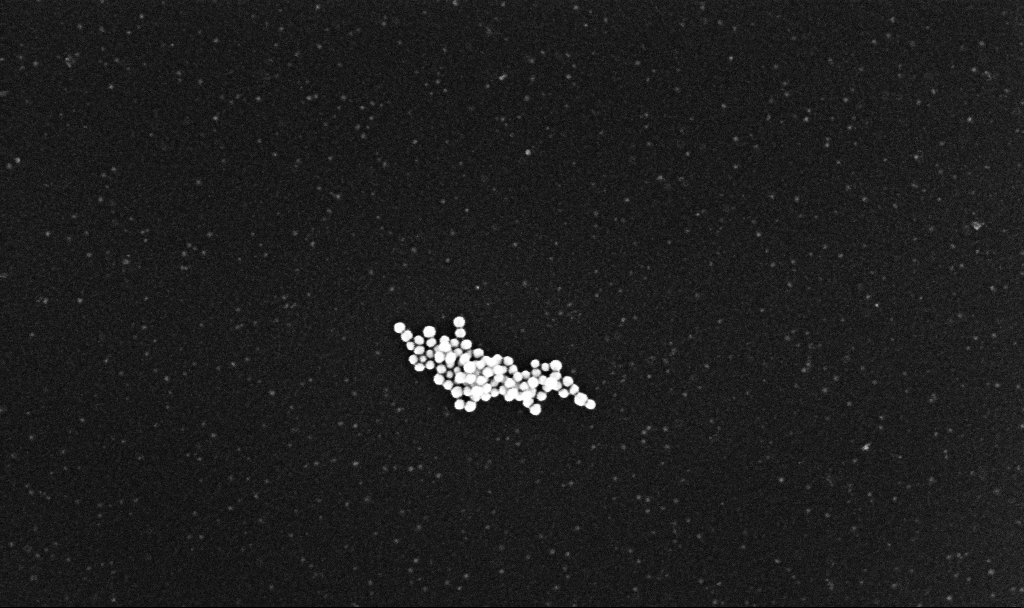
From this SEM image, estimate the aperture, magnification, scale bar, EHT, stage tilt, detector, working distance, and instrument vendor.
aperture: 30 µm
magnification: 174.24 K X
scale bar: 200 nm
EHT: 10 kV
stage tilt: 0°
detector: InLens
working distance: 3.2 mm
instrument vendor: Zeiss